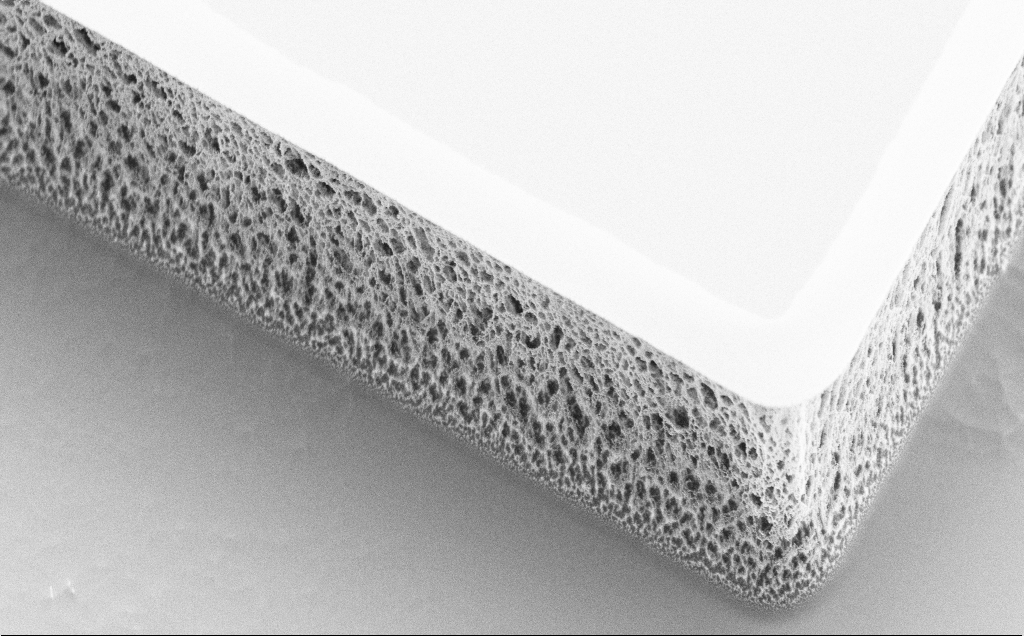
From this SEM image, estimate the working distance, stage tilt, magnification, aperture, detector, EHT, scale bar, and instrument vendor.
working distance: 8 mm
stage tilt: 45°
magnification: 6.79 K X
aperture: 30 µm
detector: SE2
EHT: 5 kV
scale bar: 10000 nm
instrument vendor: Zeiss